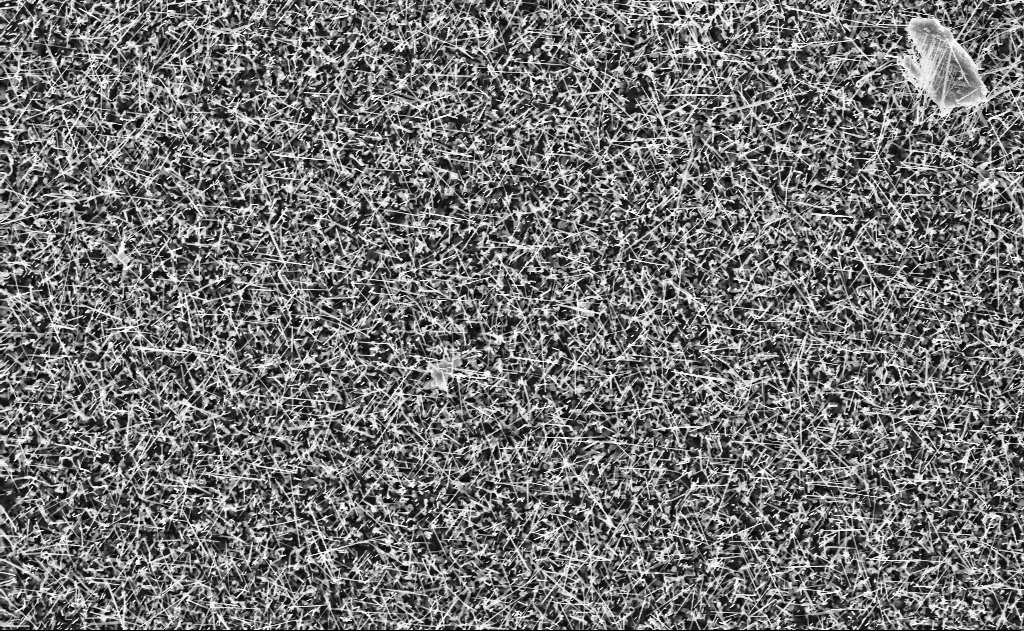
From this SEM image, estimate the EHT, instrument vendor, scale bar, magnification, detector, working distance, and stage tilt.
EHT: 10 kV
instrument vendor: Zeiss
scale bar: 2000 nm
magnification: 10 K X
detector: InLens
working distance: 10 mm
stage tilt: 0°